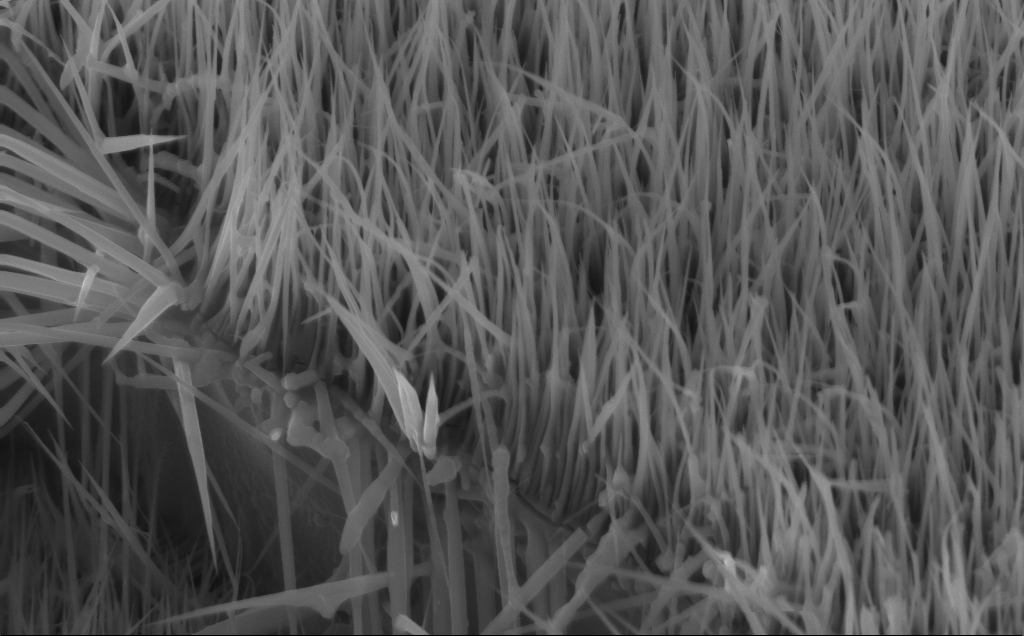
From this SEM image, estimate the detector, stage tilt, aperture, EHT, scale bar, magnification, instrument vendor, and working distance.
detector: InLens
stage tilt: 45°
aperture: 30 µm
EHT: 10 kV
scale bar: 1000 nm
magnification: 40 K X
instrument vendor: Zeiss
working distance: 5 mm